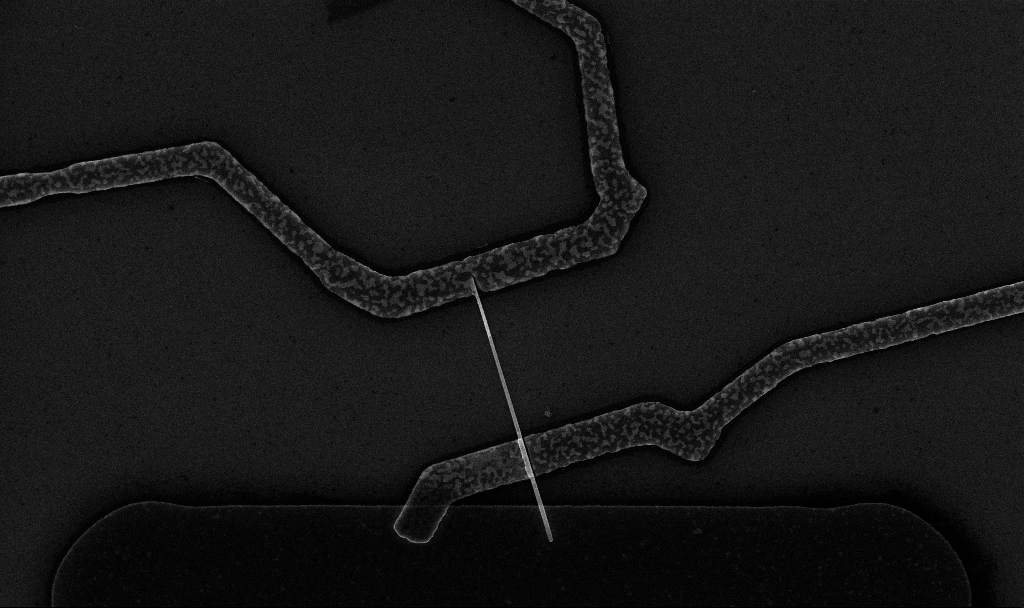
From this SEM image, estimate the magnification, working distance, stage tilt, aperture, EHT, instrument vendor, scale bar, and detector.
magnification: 16.52 K X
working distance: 6.7 mm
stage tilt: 0°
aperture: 30 µm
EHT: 10 kV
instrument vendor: Zeiss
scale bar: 2000 nm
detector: InLens